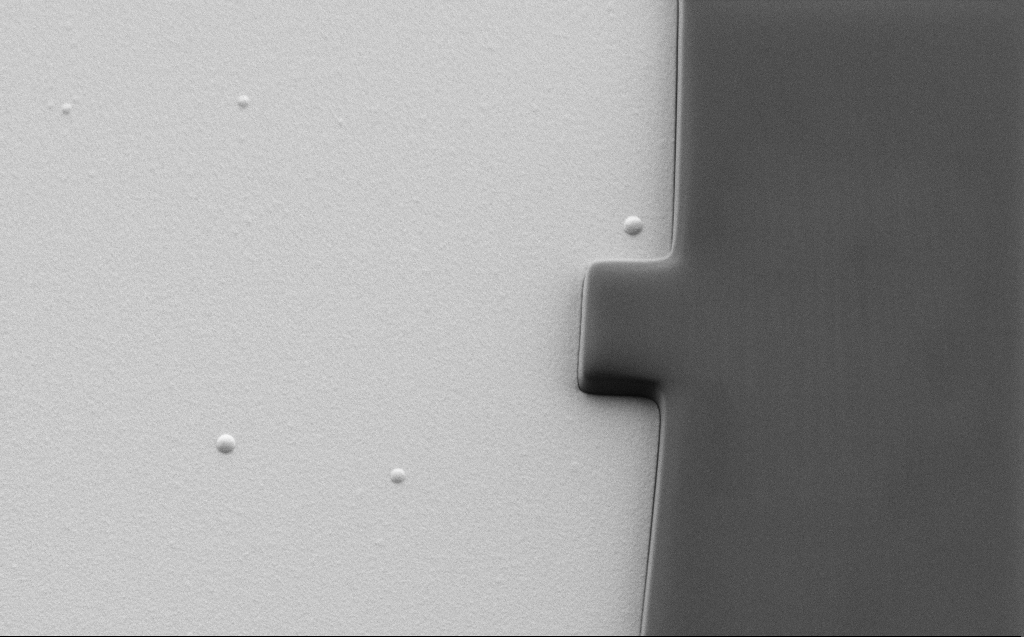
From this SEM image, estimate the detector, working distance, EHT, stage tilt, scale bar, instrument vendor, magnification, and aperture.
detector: SE2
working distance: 6 mm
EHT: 1.1 kV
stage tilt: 30°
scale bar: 10000 nm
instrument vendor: Zeiss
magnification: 5.33 K X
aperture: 30 µm